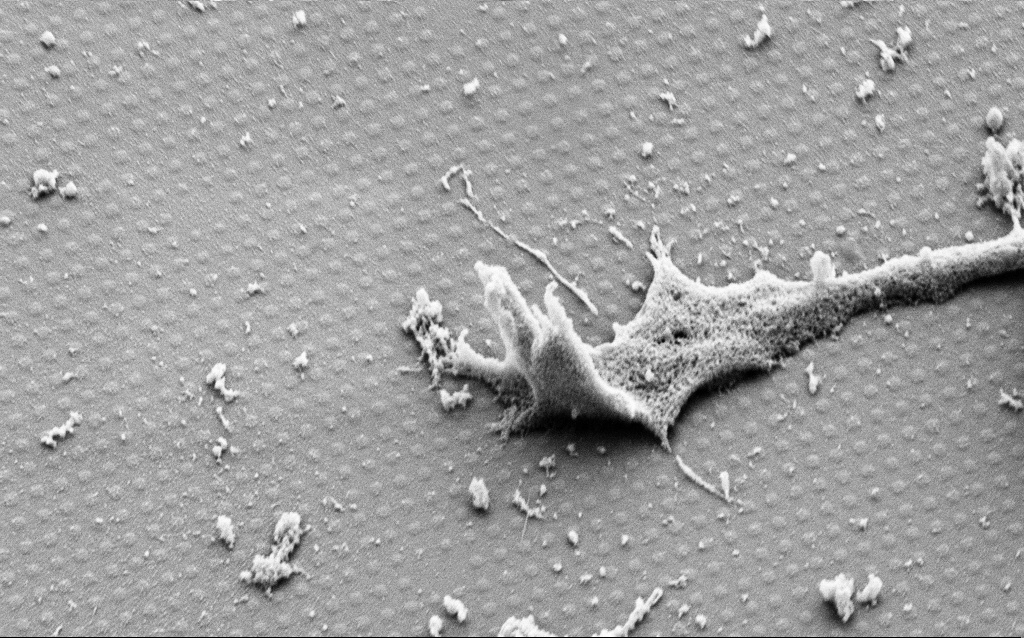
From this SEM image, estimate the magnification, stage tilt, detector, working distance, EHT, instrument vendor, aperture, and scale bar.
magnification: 18.57 K X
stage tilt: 45°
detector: SE2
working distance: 9 mm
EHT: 3 kV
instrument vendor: Zeiss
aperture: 30 µm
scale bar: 2000 nm